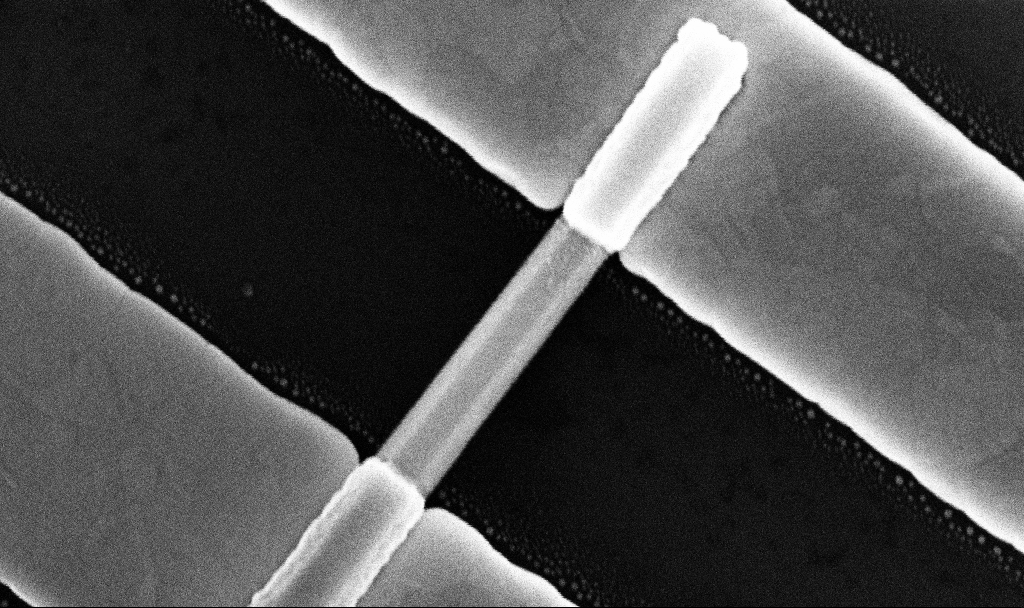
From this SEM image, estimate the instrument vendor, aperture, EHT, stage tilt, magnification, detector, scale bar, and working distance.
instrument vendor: Zeiss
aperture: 30 µm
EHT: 10 kV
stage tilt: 0°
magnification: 163.14 K X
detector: InLens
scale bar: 200 nm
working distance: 7.8 mm